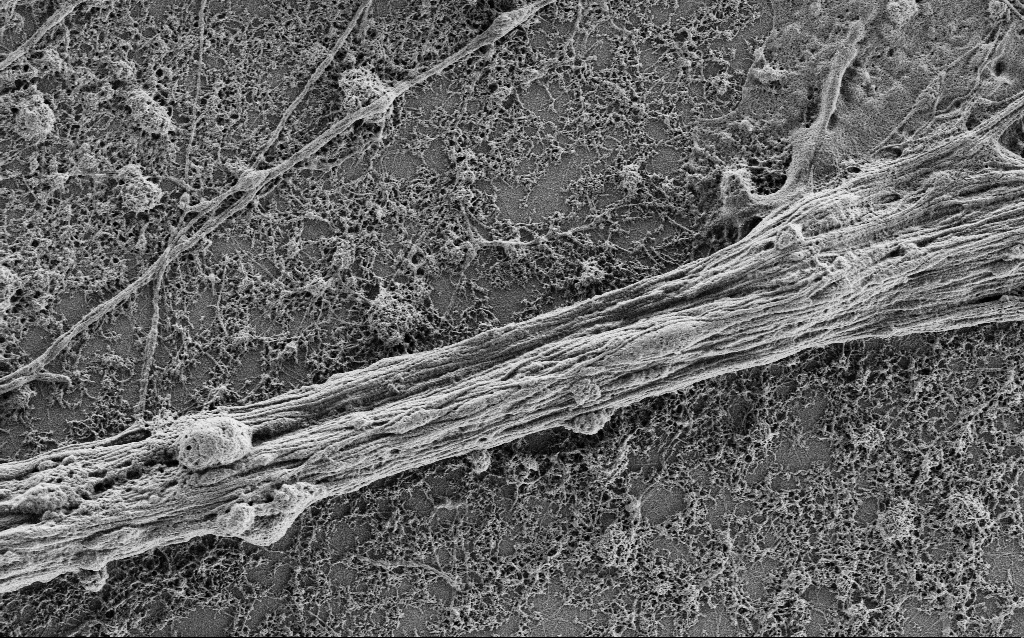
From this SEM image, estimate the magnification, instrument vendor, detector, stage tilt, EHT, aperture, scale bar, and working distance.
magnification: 10 K X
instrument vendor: Zeiss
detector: SE2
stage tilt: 0°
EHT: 1 kV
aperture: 30 µm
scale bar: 2000 nm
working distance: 4 mm